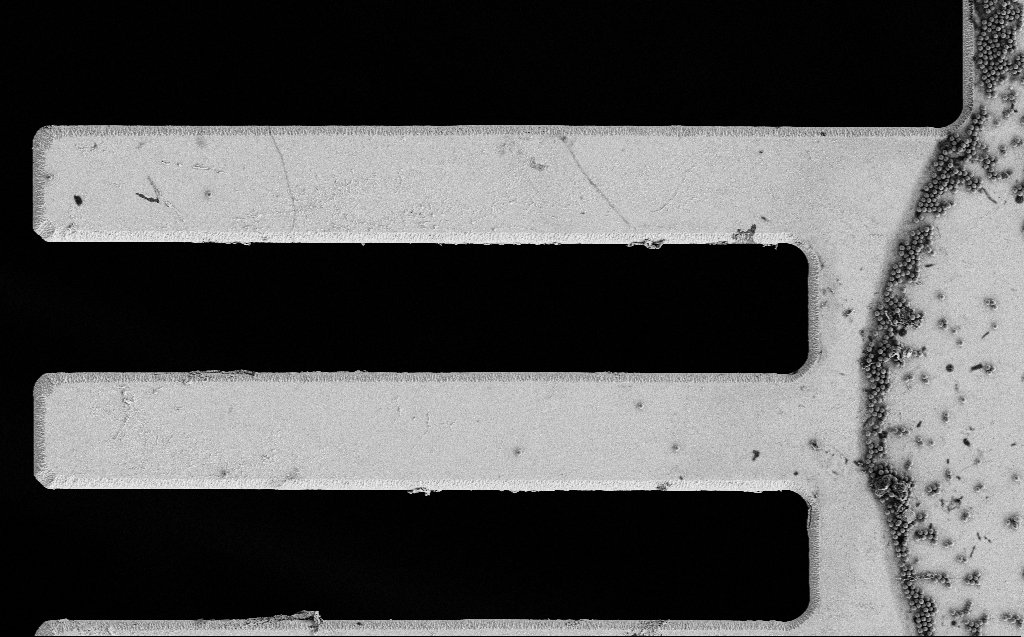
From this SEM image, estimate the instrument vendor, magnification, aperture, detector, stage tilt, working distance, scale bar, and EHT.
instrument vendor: Zeiss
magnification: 2.28 K X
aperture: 30 µm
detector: SE2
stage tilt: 0°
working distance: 7 mm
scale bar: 20000 nm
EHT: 3 kV